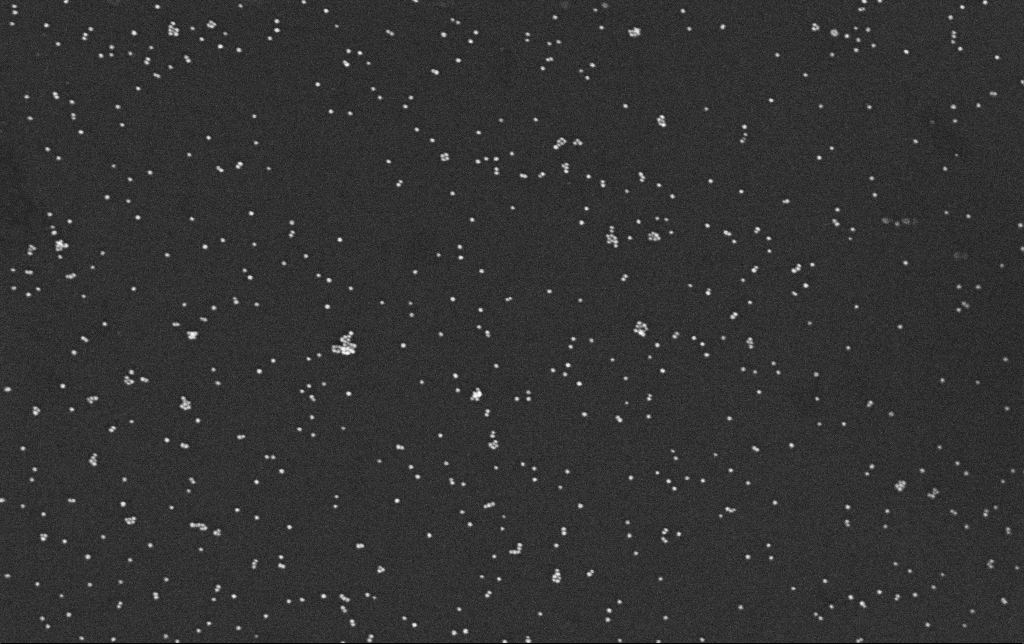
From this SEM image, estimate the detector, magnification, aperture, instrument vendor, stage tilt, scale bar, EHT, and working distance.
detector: InLens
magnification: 100 K X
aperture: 30 µm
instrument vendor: Zeiss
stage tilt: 0°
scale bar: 200 nm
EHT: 10 kV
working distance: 3.4 mm